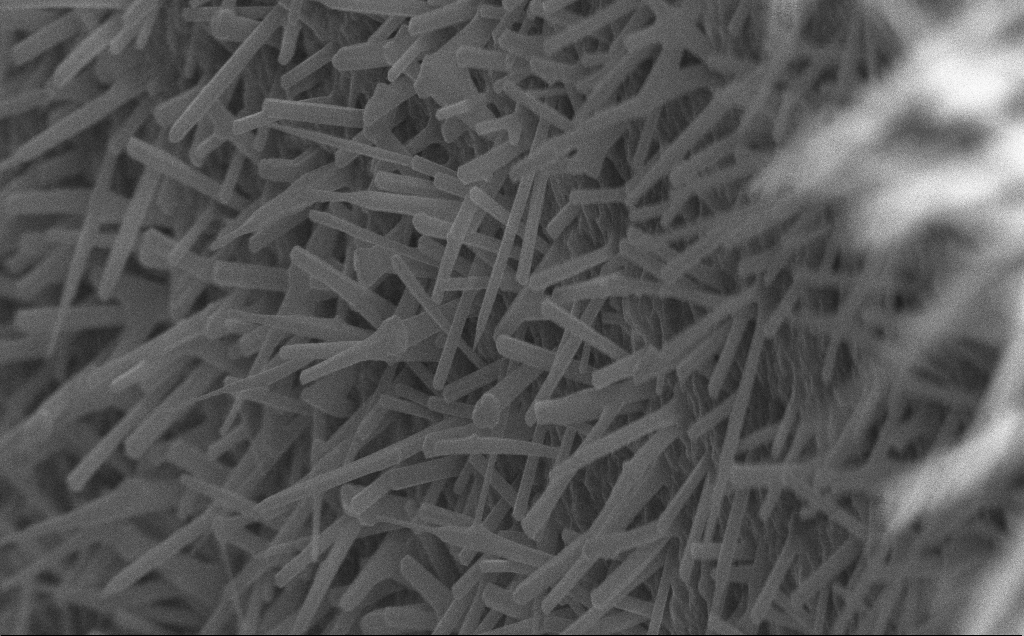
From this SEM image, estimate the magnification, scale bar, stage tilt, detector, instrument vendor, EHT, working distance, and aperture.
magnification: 43.75 K X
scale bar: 1000 nm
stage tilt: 0°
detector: InLens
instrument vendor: Zeiss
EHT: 10 kV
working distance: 7 mm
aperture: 30 µm